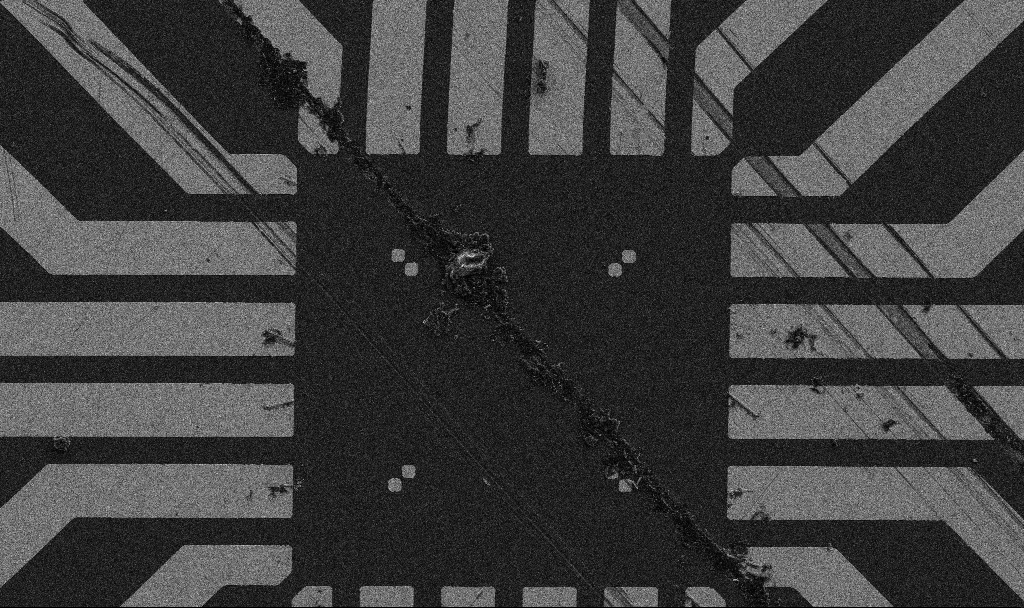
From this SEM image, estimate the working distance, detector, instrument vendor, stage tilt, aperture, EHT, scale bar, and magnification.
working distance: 9 mm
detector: SE2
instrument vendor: Zeiss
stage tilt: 0°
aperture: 30 µm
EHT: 5 kV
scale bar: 20000 nm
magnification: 1 K X